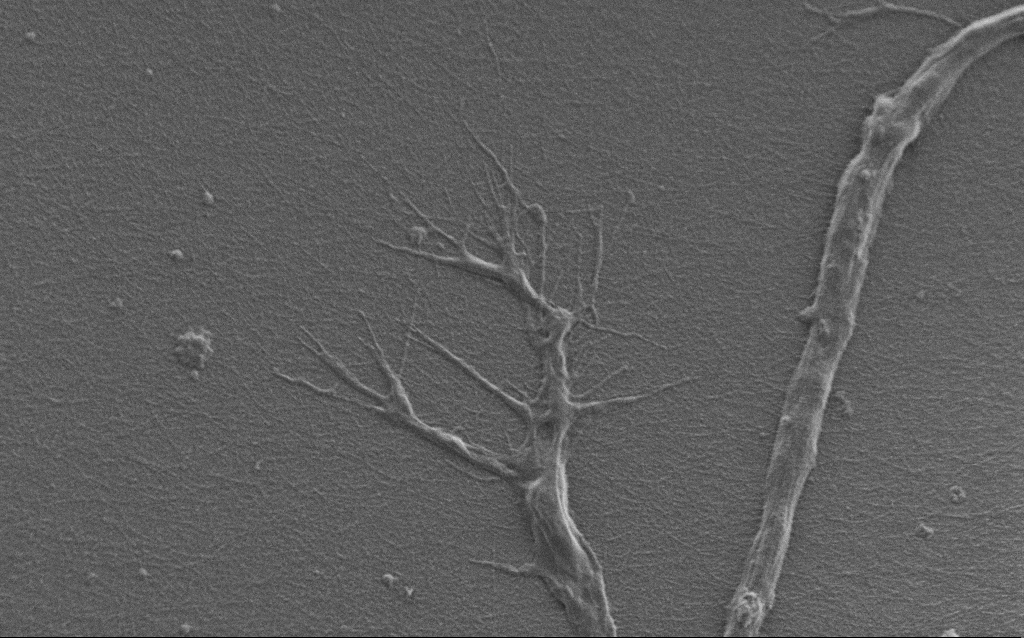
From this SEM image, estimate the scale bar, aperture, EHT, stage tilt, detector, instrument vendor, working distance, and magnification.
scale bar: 2000 nm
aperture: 30 µm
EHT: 0.9 kV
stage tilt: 0°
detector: SE2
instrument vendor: Zeiss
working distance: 7 mm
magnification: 7.5 K X